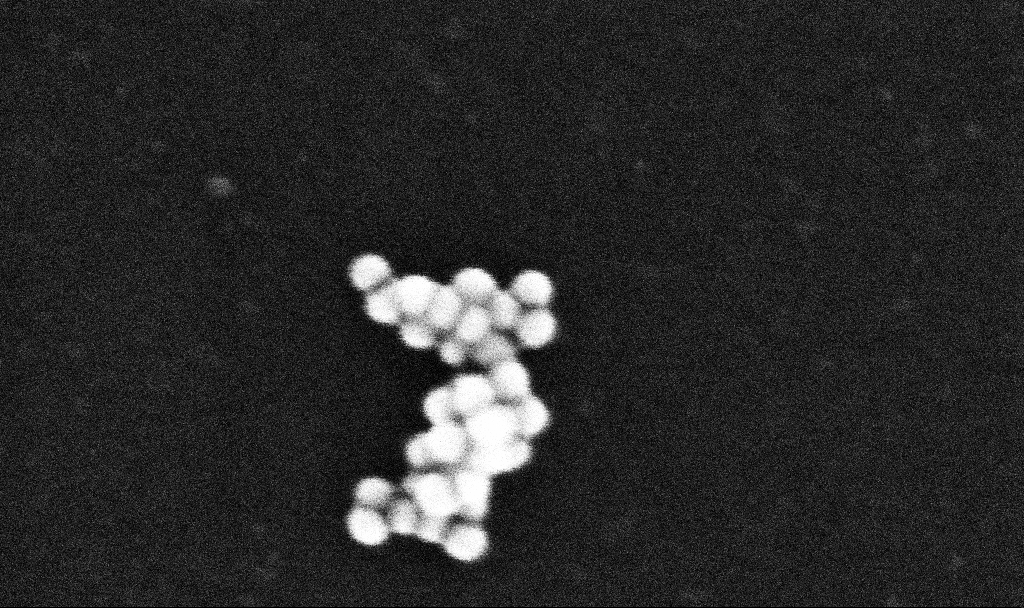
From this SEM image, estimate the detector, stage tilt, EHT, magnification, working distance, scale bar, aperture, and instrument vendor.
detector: InLens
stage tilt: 0°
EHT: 10 kV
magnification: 625.57 K X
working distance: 3.1 mm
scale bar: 100 nm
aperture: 30 µm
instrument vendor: Zeiss